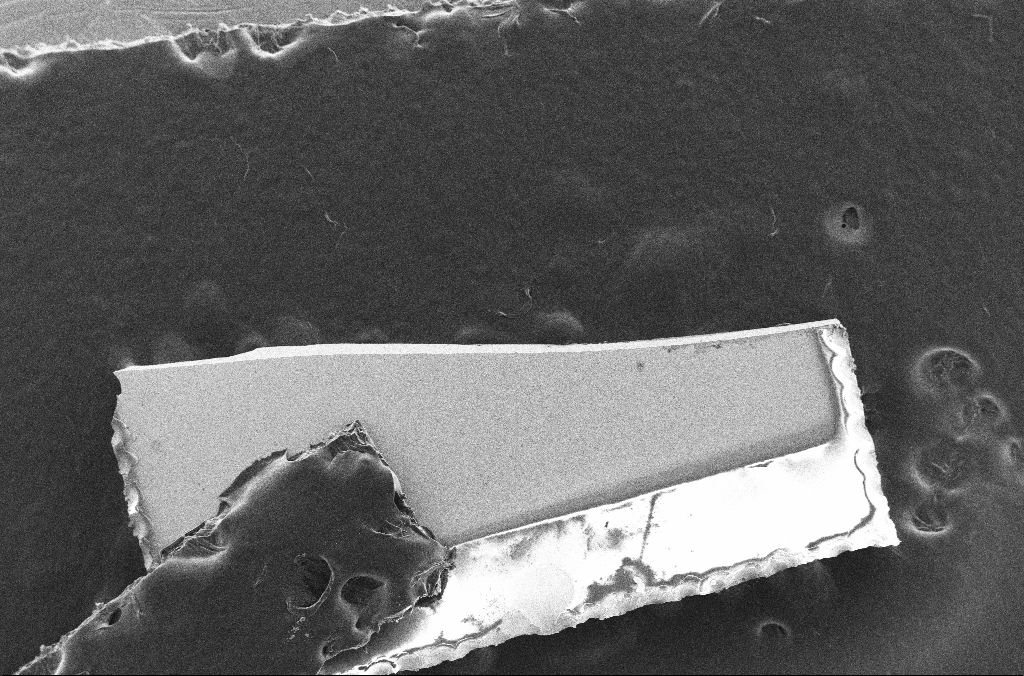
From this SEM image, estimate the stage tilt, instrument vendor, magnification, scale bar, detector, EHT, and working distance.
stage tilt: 0.1°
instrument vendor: Zeiss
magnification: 0.09 K X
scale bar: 200000 nm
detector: SE2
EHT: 10 kV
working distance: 5.2 mm